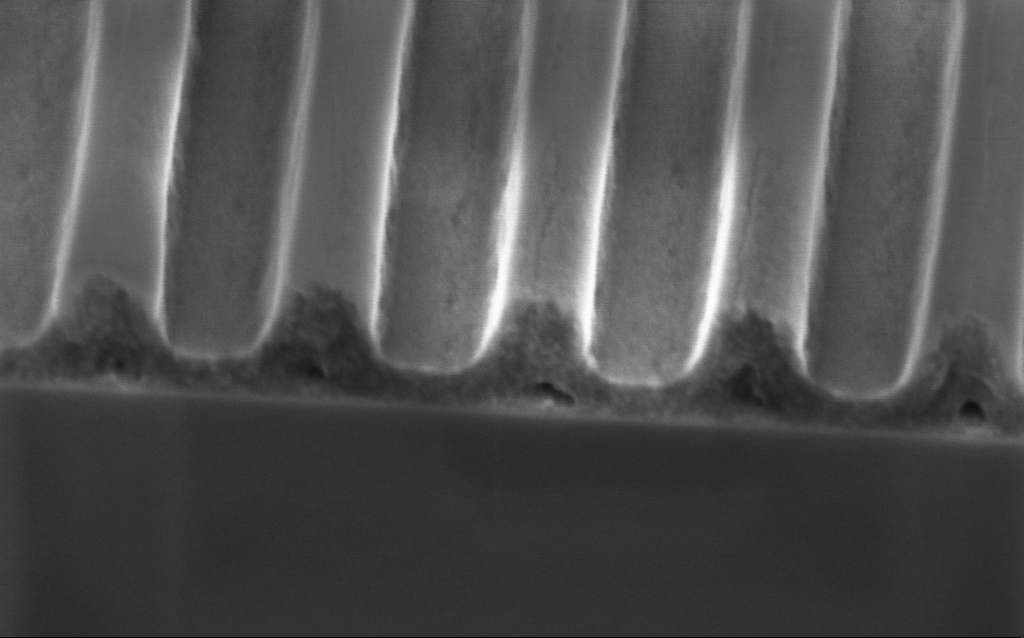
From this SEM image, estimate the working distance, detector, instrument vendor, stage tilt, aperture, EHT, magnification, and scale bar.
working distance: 3.6 mm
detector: InLens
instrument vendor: Zeiss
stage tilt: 45°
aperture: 30 µm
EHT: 2 kV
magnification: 158.79 K X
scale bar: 200 nm